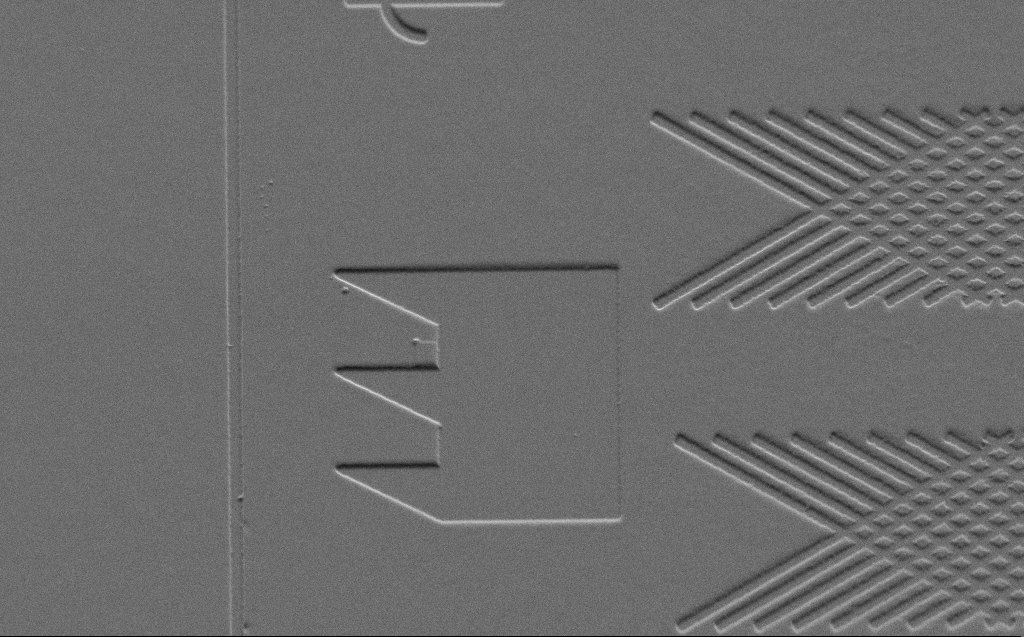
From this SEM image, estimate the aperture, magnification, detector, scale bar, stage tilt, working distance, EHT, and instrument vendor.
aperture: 30 µm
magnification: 2.84 K X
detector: SE2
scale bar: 10000 nm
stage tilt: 45°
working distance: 6 mm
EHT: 3 kV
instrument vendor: Zeiss